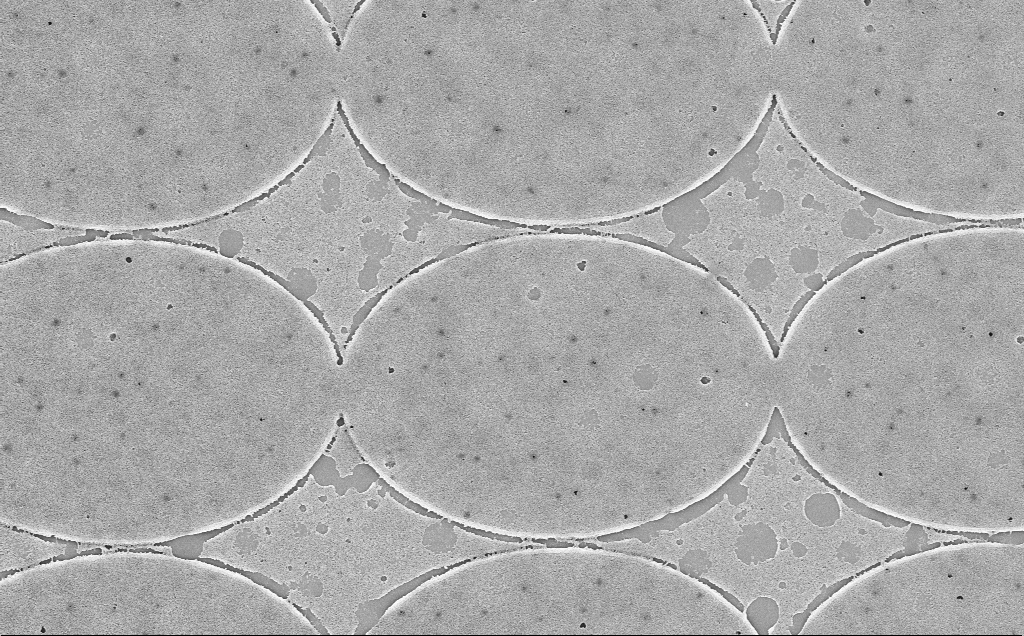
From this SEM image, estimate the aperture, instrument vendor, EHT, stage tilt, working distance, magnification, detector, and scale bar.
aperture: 30 µm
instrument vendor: Zeiss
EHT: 5 kV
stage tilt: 0°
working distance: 6 mm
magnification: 16.05 K X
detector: InLens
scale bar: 2000 nm